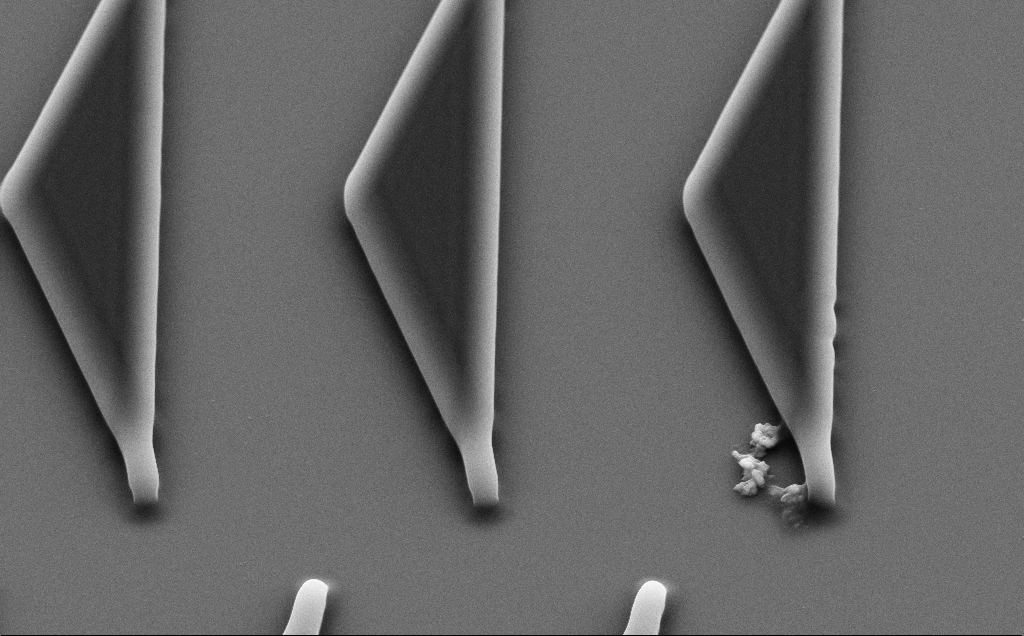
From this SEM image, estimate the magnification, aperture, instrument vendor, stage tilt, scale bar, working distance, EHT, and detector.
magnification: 7.35 K X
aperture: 30 µm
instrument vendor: Zeiss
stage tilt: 35°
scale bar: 2000 nm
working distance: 8 mm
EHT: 10 kV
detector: SE2